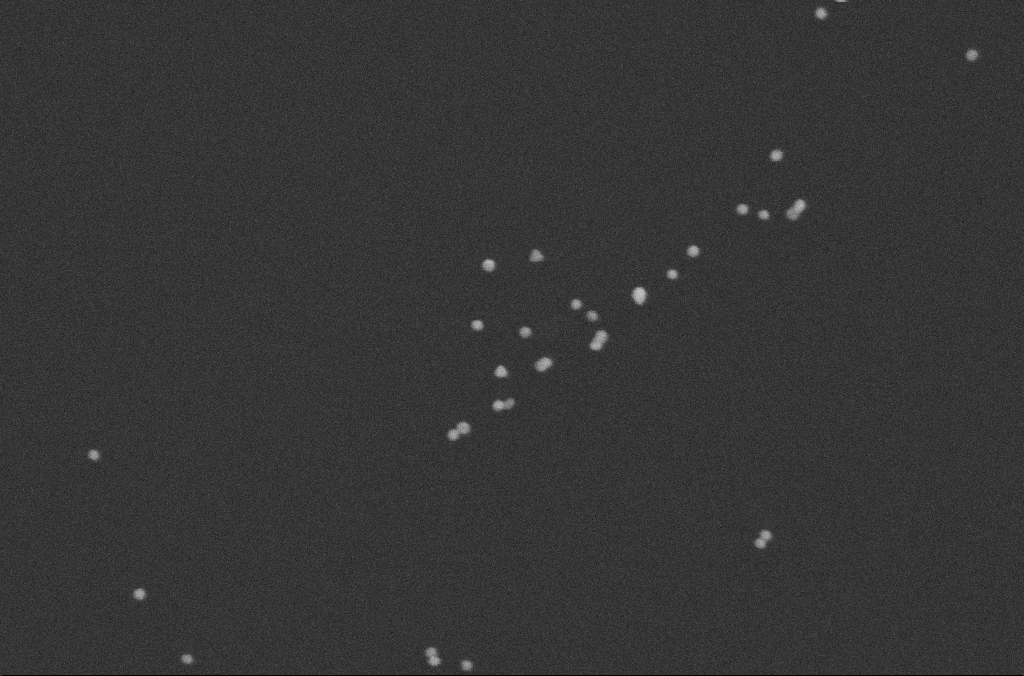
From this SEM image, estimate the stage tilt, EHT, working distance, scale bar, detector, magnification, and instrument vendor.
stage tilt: -0°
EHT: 10 kV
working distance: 2.5 mm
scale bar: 100 nm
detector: SE2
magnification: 217.51 K X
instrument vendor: Zeiss